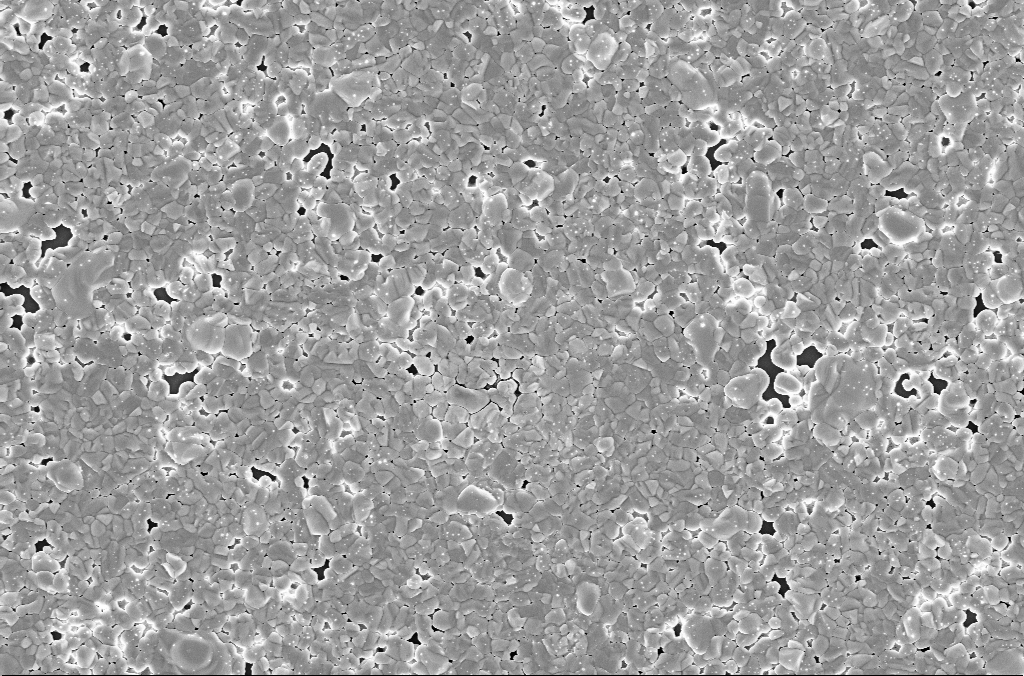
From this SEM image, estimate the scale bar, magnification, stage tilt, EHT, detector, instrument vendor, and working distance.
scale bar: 2000 nm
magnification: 10 K X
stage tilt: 0°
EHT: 5 kV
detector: InLens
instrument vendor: Zeiss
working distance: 3.1 mm